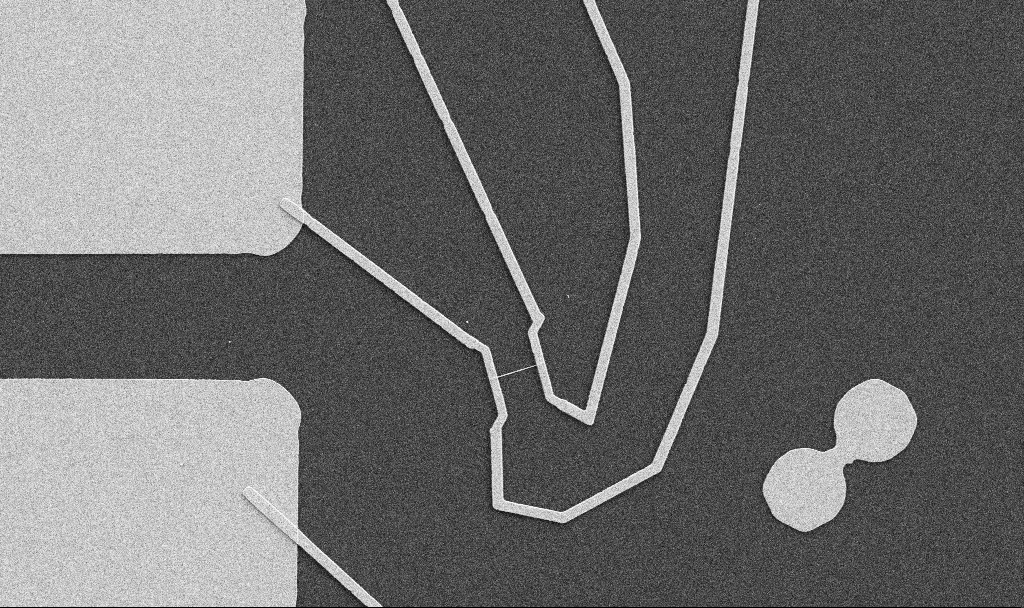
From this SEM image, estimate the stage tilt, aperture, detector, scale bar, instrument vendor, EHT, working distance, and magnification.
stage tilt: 0°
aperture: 30 µm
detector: SE2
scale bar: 10000 nm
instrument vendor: Zeiss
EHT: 5 kV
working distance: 10.7 mm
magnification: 5 K X